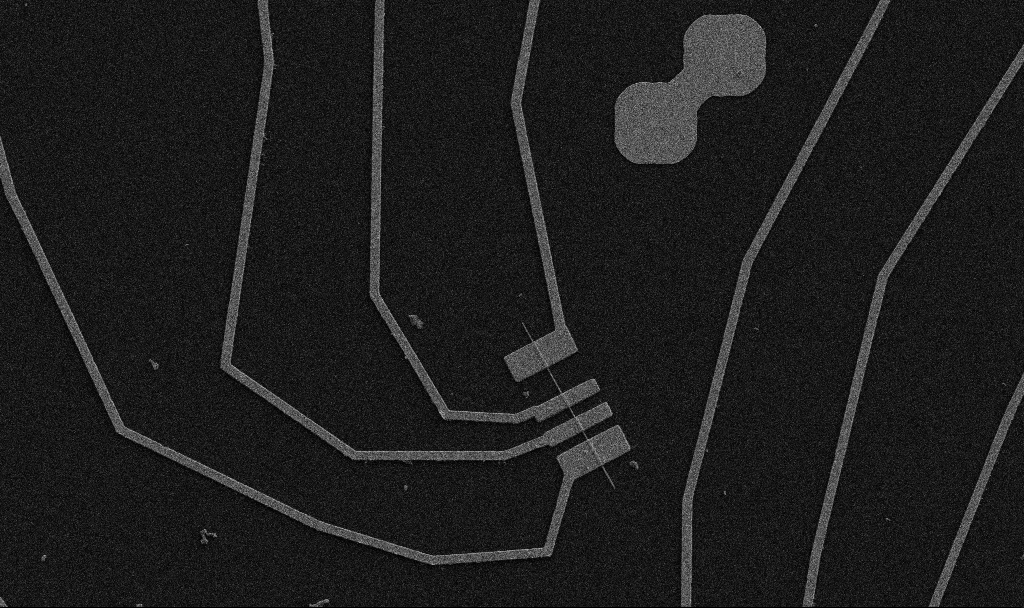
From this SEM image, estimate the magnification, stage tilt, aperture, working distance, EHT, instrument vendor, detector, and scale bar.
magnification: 5 K X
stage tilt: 0°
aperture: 30 µm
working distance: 10.7 mm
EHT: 5 kV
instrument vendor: Zeiss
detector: SE2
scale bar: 10000 nm